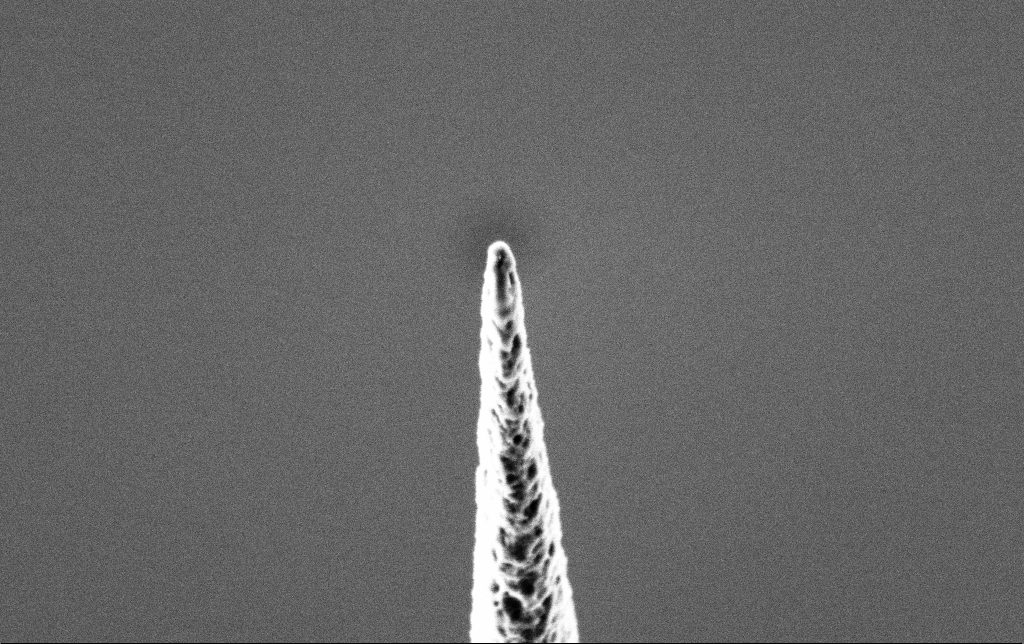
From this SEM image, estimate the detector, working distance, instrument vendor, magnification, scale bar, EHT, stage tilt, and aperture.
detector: SE2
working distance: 7.4 mm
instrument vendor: Zeiss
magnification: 50 K X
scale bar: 1000 nm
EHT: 2 kV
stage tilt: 45°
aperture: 30 µm